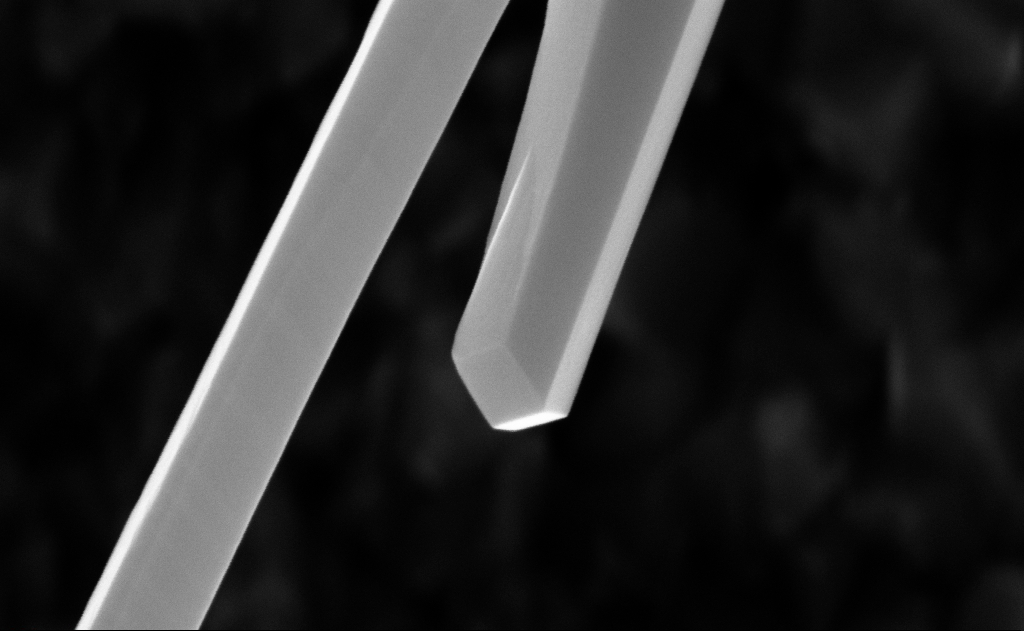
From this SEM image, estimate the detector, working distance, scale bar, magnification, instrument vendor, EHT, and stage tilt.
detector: InLens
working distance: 6 mm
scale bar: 200 nm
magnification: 150 K X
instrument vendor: Zeiss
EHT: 10 kV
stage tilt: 0°